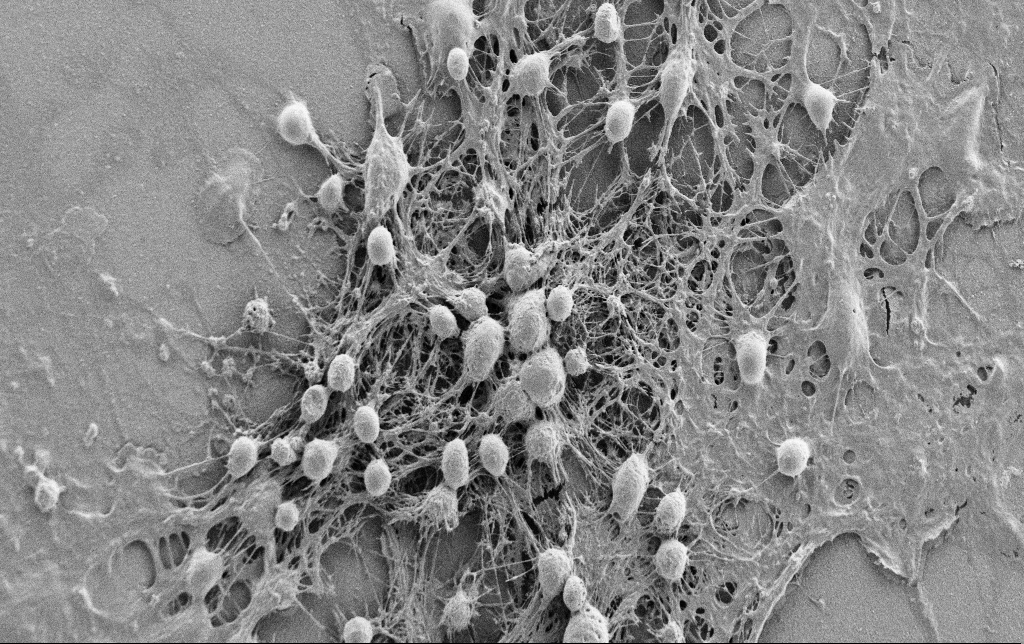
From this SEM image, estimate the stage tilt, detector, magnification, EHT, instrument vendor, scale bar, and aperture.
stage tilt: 0°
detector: SE2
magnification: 2.5 K X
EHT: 0.9 kV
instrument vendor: Zeiss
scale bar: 20000 nm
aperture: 30 µm